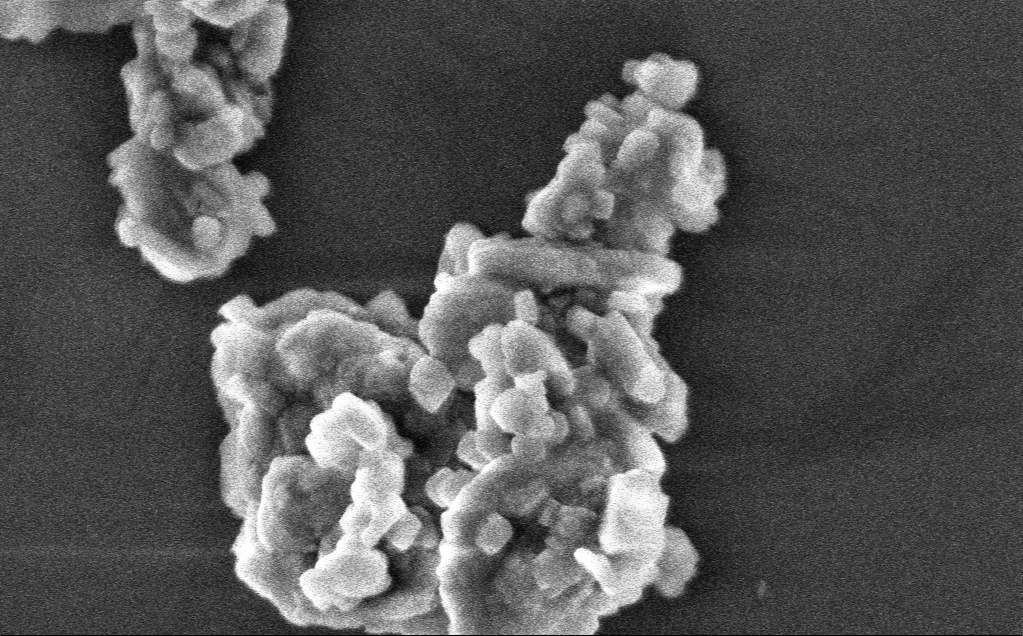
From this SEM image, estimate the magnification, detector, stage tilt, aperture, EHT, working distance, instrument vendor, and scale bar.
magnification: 122.33 K X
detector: InLens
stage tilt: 0°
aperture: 30 µm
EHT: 3 kV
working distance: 3 mm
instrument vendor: Zeiss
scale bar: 200 nm